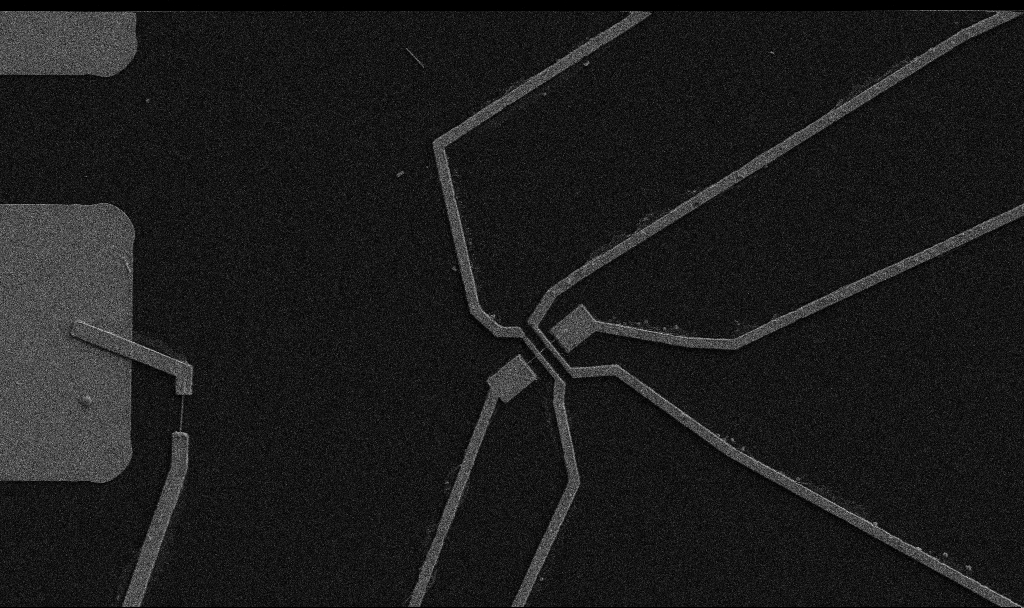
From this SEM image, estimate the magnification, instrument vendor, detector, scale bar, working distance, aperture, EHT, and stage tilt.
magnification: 5 K X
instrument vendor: Zeiss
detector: SE2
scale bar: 10000 nm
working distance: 10.7 mm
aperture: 30 µm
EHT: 5 kV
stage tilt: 0°